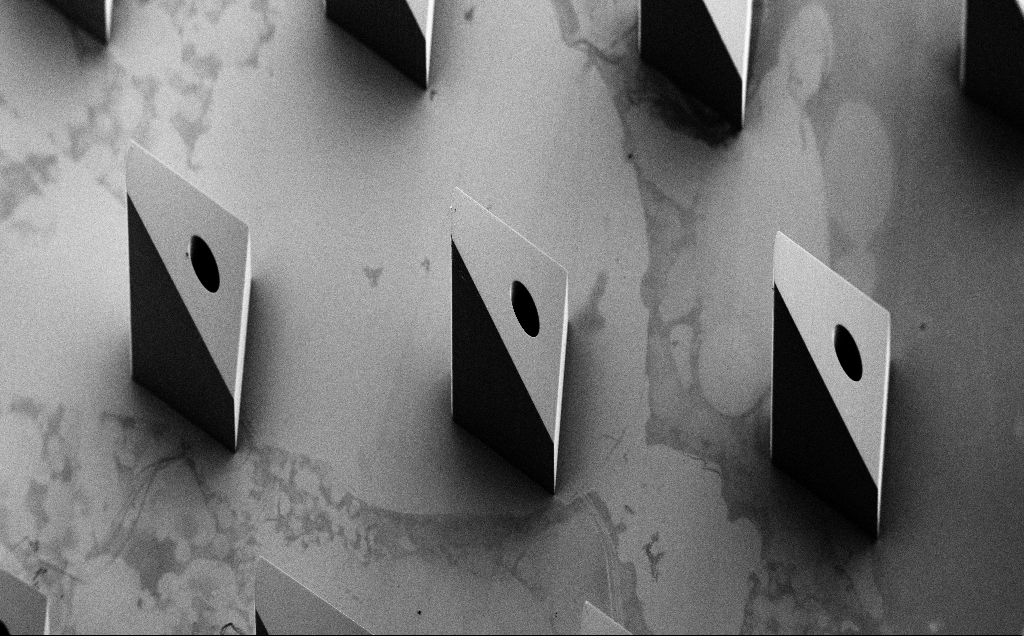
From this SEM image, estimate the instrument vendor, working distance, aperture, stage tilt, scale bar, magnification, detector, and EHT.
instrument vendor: Zeiss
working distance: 8 mm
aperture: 30 µm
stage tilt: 35°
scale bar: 200000 nm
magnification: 0.121 K X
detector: SE2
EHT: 5 kV